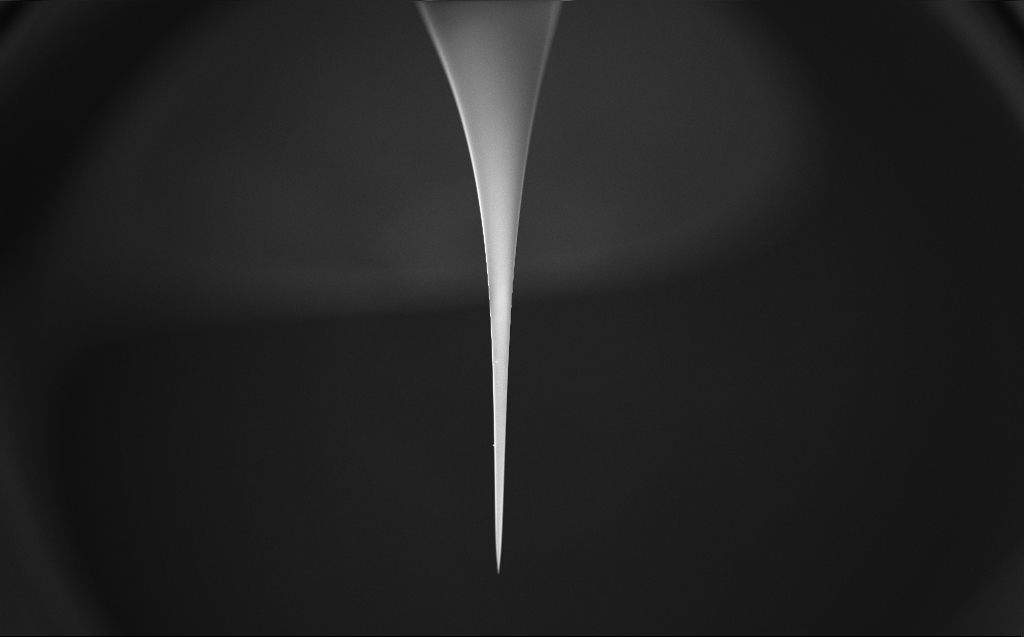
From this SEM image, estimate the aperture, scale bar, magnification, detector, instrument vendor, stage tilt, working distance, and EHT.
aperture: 30 µm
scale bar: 200000 nm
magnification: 0.08 K X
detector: InLens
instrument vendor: Zeiss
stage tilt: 45.1°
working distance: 7 mm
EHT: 2 kV